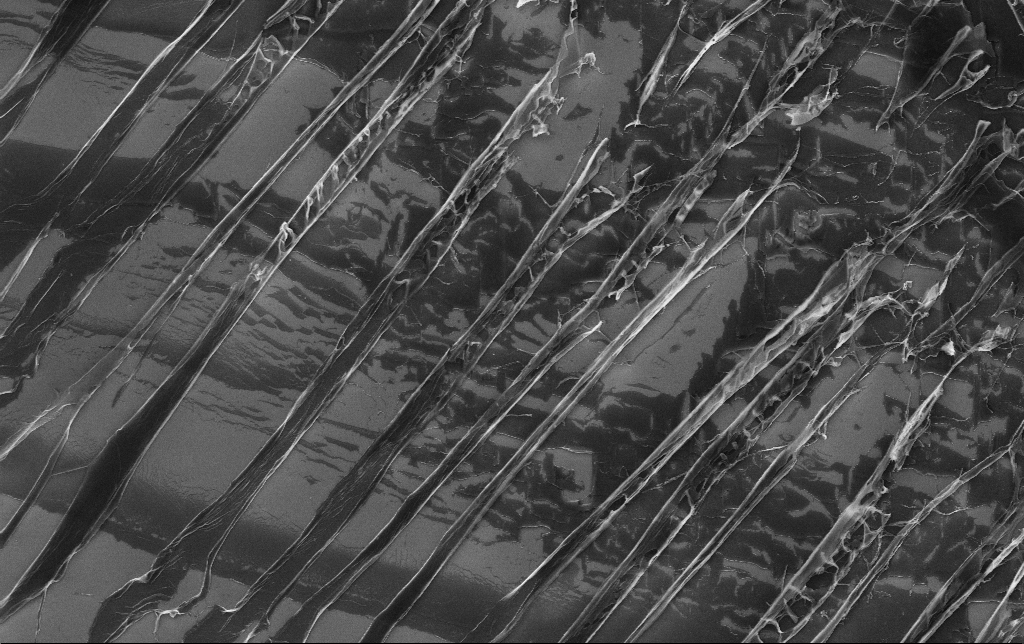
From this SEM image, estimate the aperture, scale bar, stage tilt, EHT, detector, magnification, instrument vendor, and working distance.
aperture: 30 µm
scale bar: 10000 nm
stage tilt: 0°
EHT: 6 kV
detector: InLens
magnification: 4.23 K X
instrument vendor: Zeiss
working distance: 3.1 mm